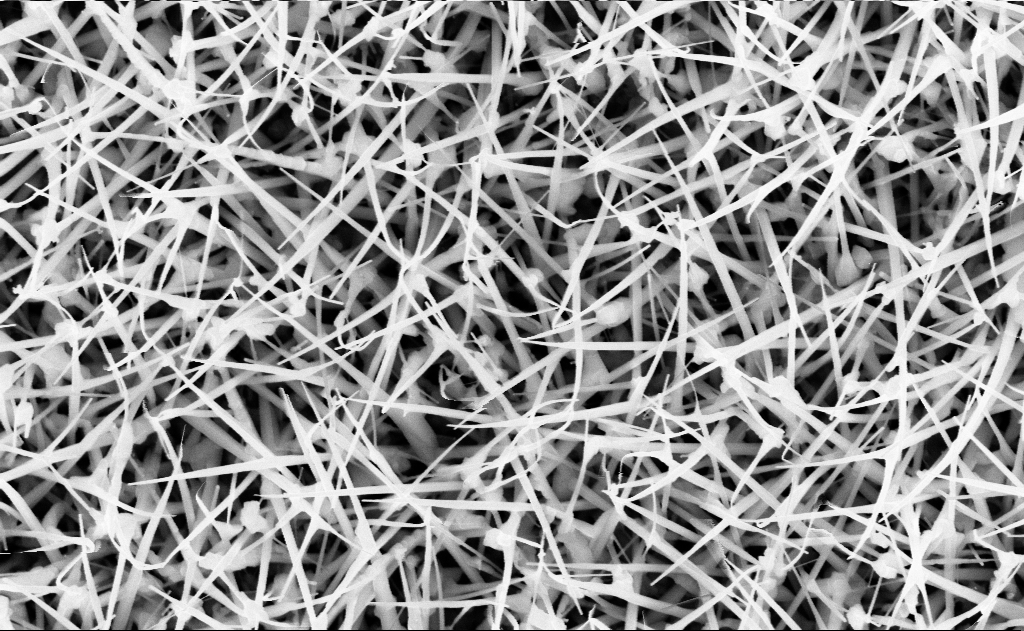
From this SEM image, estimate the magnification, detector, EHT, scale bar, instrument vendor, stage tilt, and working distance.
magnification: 40 K X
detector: InLens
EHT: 10 kV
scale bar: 1000 nm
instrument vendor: Zeiss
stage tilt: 0°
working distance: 13 mm